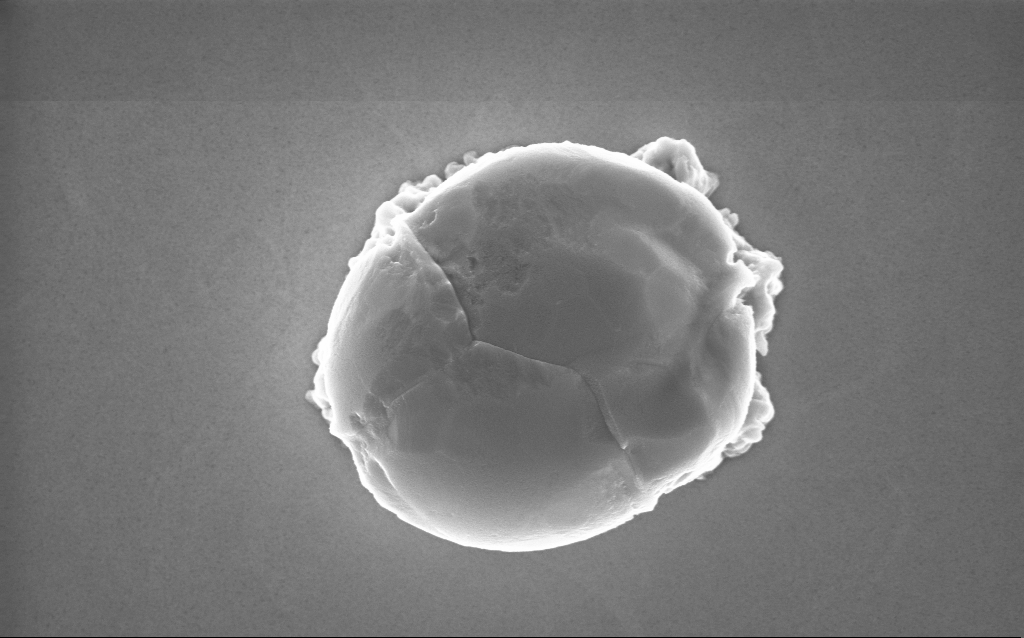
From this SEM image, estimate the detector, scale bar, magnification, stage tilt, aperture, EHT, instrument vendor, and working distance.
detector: InLens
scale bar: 1000 nm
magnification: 52.13 K X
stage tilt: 0°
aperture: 30 µm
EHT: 10 kV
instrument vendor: Zeiss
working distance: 2 mm